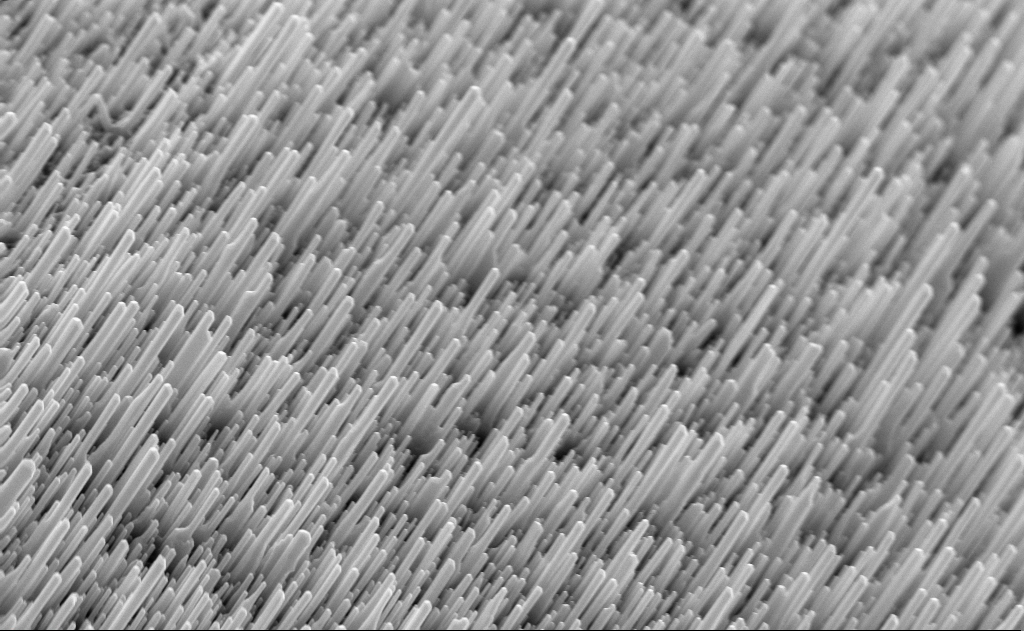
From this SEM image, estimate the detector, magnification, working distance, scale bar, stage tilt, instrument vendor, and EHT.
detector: InLens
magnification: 20 K X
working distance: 6 mm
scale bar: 2000 nm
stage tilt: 0°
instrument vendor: Zeiss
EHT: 10 kV